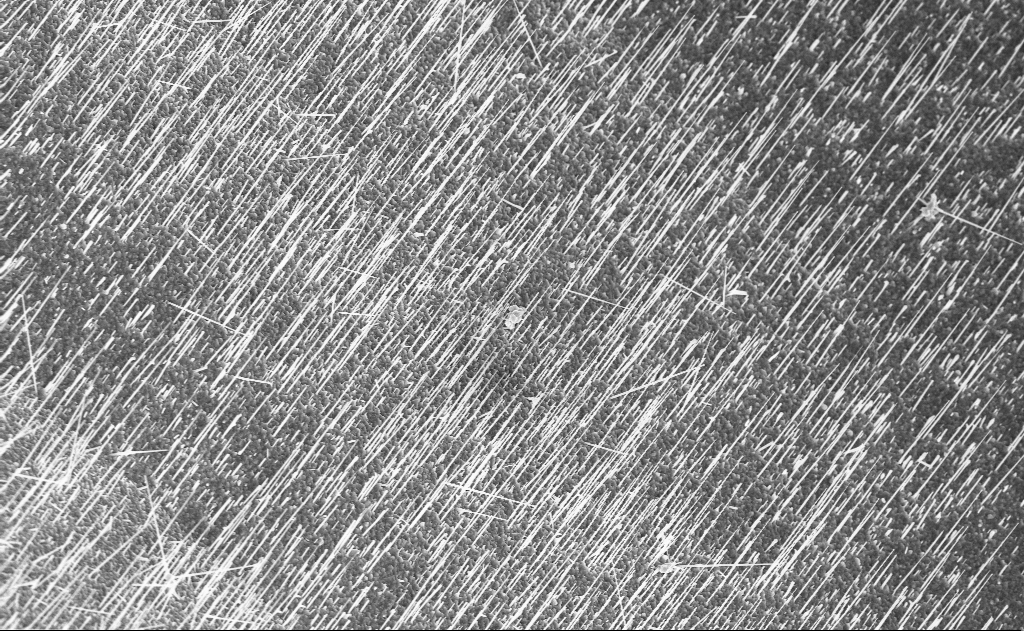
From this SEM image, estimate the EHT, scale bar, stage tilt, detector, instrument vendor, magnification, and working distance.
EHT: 10 kV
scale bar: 10000 nm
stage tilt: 0°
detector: InLens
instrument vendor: Zeiss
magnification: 5 K X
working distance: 10 mm